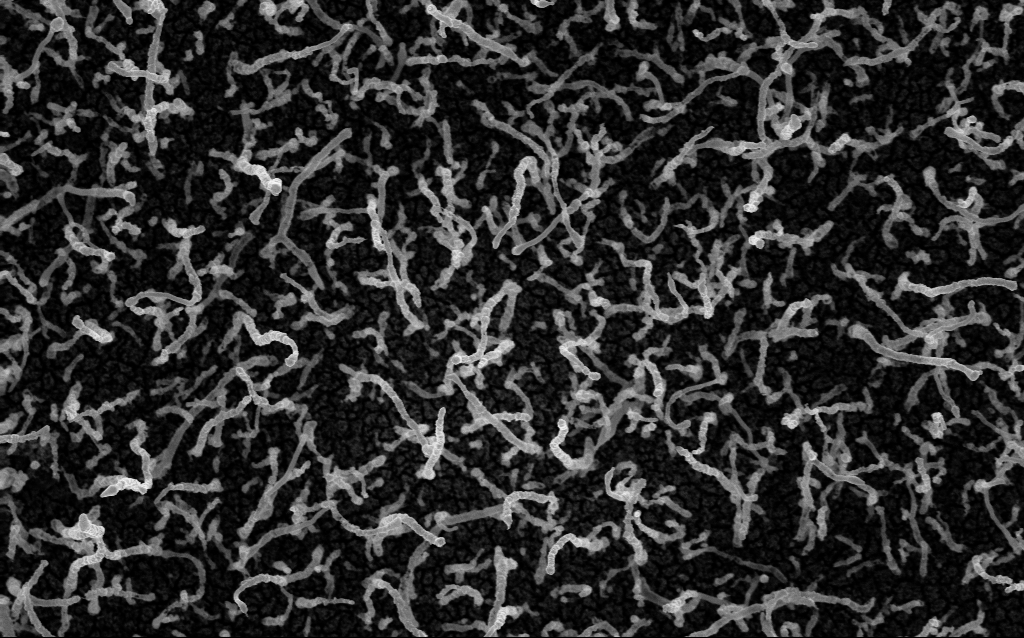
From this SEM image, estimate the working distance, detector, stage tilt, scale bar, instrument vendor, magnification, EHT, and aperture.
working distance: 2.2 mm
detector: InLens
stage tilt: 0°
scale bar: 1000 nm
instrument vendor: Zeiss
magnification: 50 K X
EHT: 5 kV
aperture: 30 µm